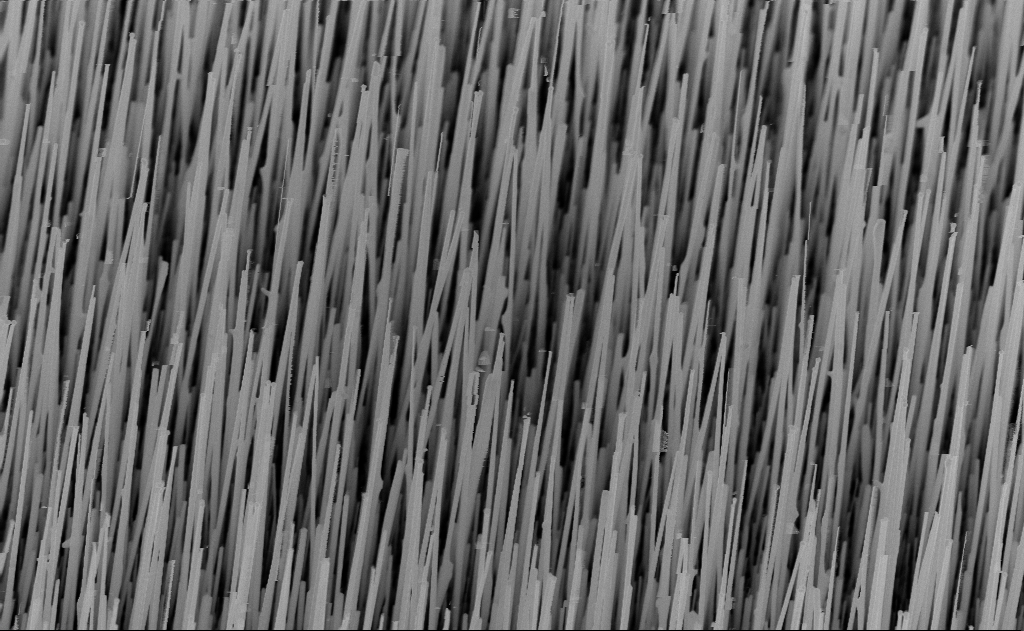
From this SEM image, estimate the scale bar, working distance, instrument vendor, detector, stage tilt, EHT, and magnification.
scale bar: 1000 nm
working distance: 9 mm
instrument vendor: Zeiss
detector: InLens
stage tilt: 30°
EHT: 10 kV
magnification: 20 K X